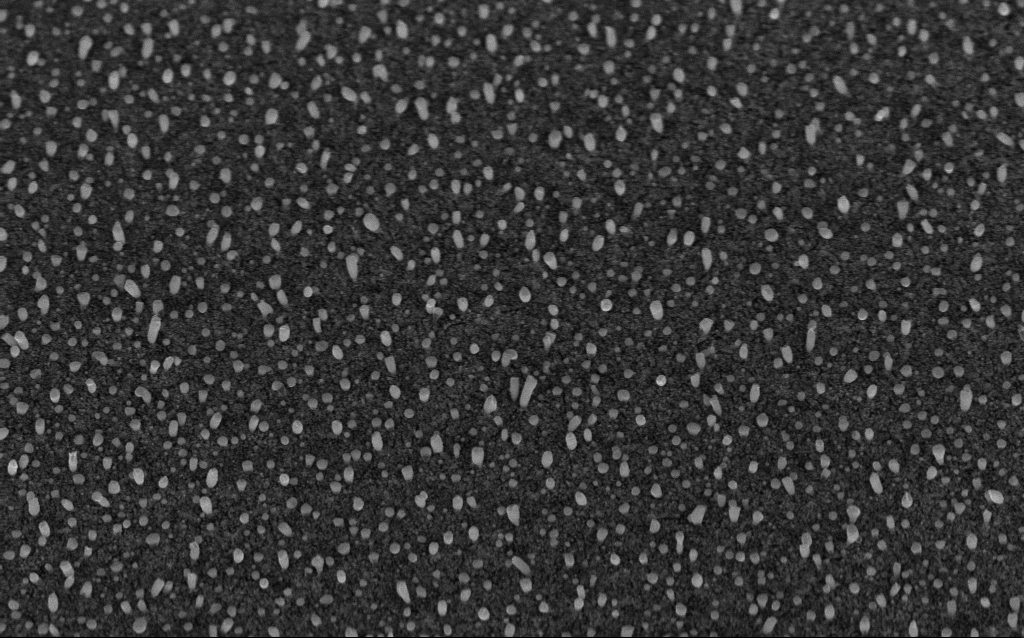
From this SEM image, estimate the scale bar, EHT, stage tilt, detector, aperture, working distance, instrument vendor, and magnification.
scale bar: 1000 nm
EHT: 5 kV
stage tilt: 45°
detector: InLens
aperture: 30 µm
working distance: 5.4 mm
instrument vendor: Zeiss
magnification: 50 K X